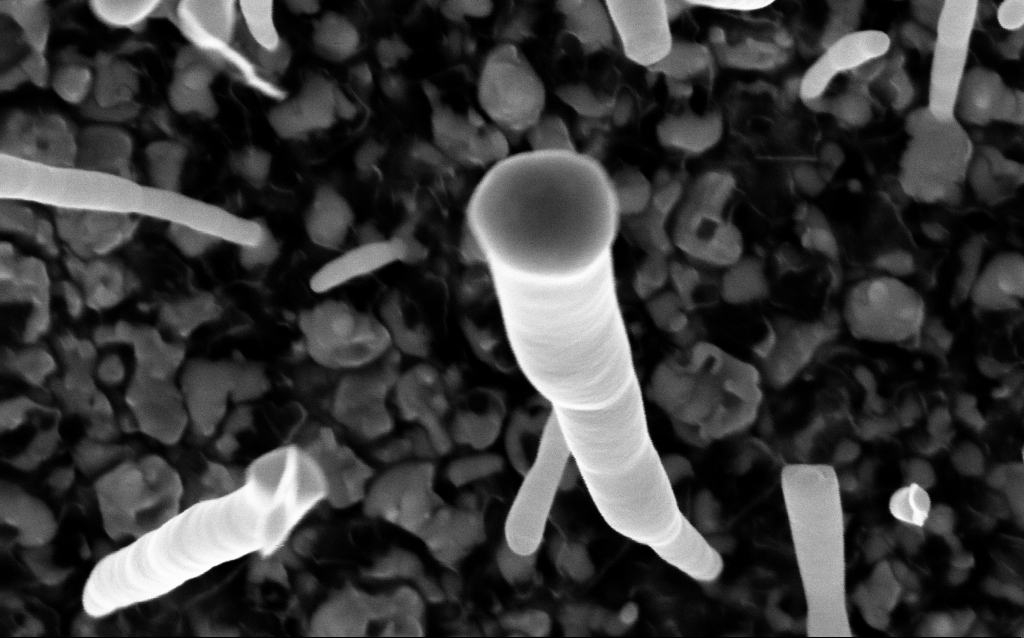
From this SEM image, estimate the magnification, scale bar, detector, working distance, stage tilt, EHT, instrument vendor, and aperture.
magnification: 200 K X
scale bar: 100 nm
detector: InLens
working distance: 2.4 mm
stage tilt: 0°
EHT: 5 kV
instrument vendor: Zeiss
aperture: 30 µm